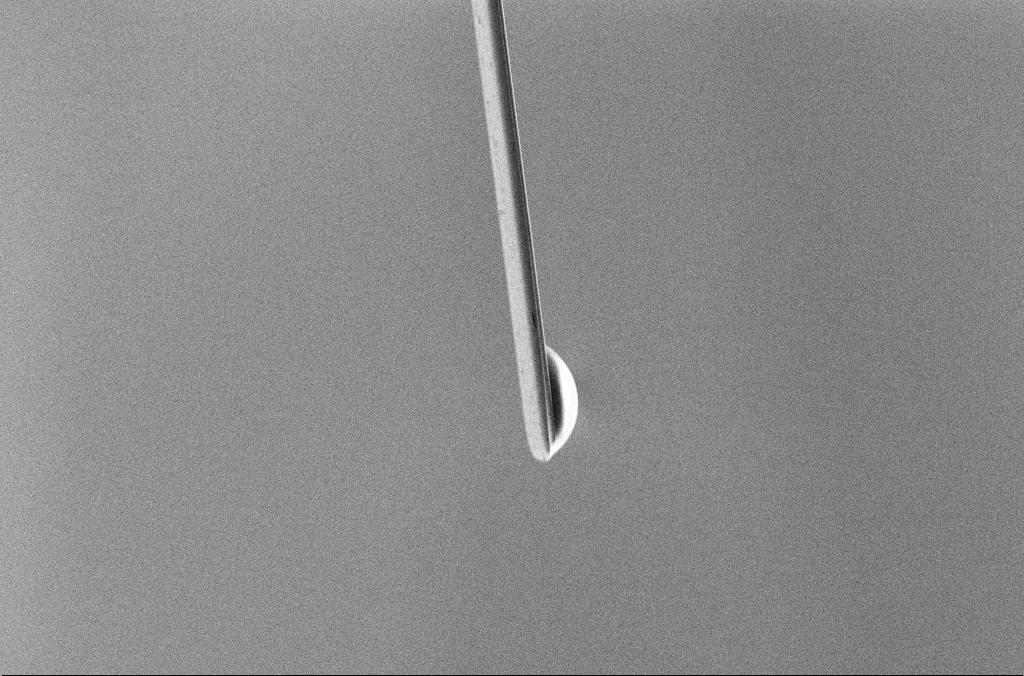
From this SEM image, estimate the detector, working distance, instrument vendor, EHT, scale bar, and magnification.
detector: SE2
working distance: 5.3 mm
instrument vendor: Zeiss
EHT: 5 kV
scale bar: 10000 nm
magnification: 2.5 K X